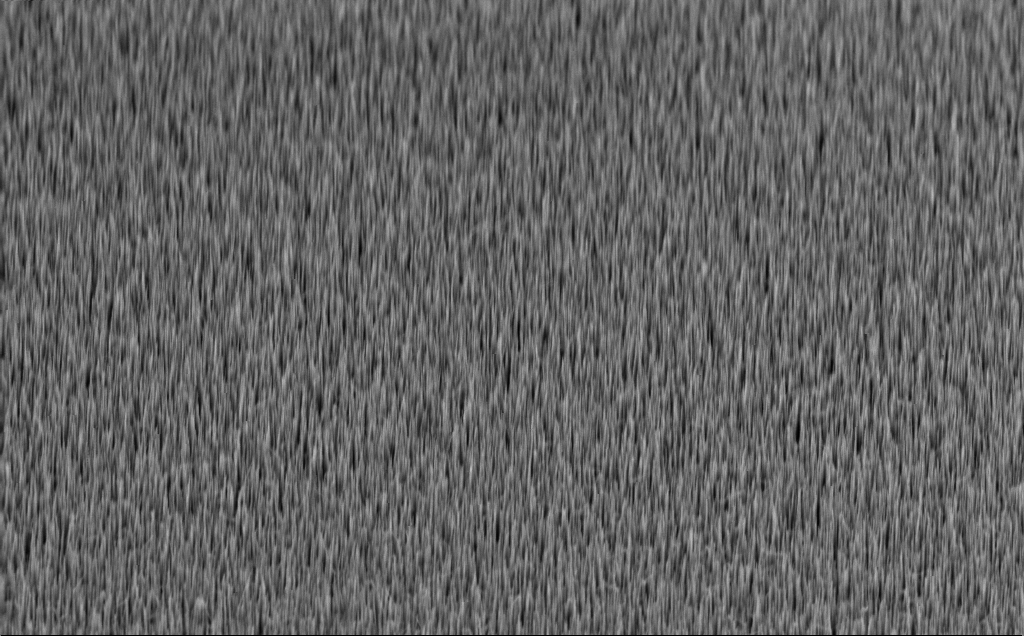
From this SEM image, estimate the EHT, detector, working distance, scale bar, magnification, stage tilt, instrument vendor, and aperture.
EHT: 10 kV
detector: InLens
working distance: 5 mm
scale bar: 1000 nm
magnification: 20 K X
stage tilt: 45°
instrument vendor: Zeiss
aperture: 30 µm